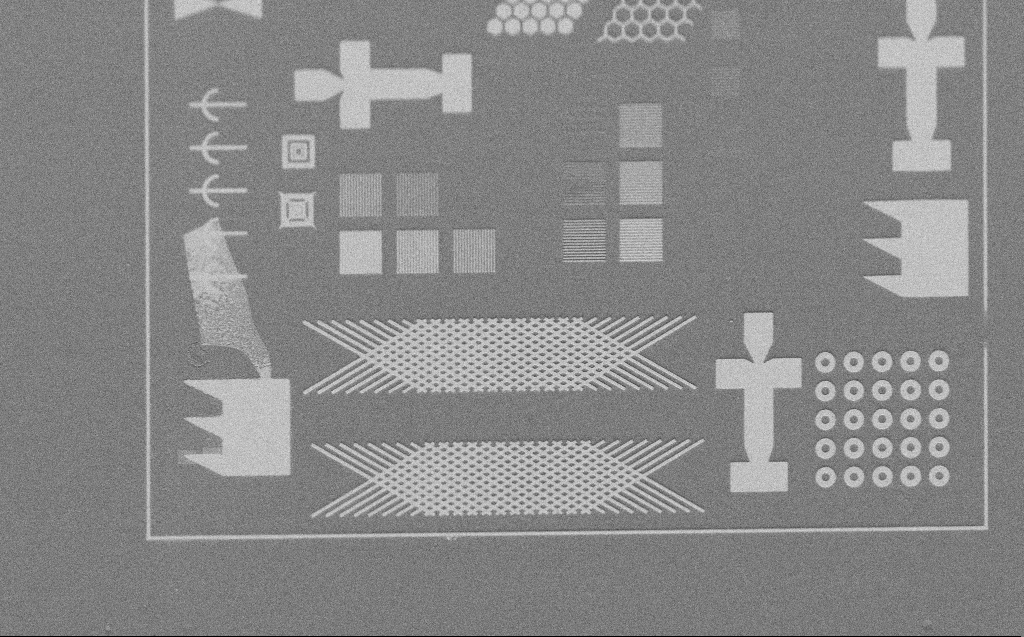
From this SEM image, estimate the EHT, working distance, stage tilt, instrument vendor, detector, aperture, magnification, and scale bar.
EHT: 2 kV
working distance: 5 mm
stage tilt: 45°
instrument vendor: Zeiss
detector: SE2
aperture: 30 µm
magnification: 1.05 K X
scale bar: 20000 nm